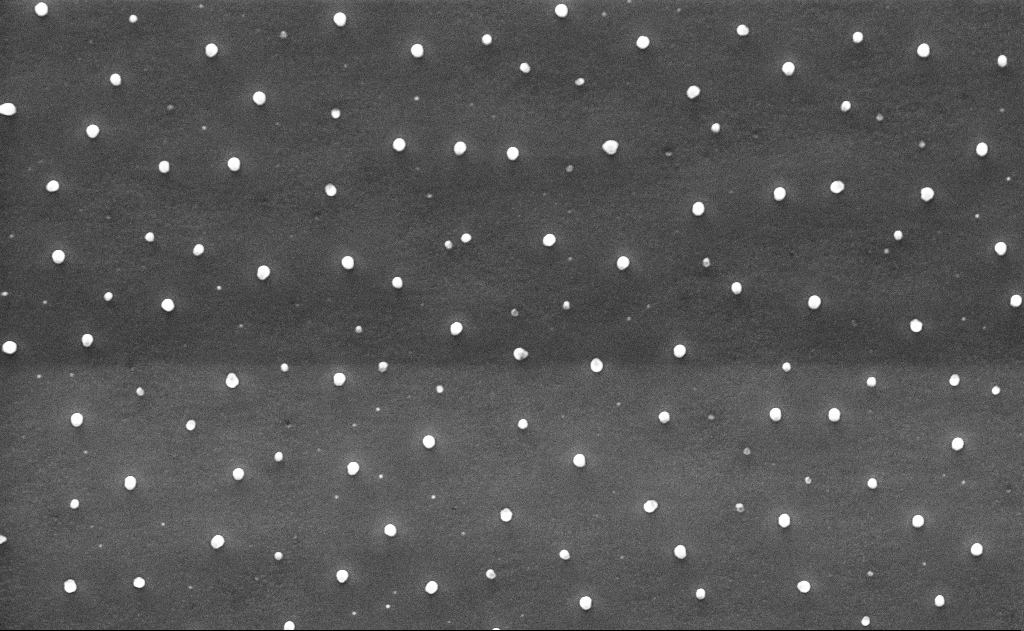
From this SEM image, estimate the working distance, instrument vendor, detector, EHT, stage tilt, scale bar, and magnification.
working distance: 10 mm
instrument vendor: Zeiss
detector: InLens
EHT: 10 kV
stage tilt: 0°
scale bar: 2000 nm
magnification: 10 K X